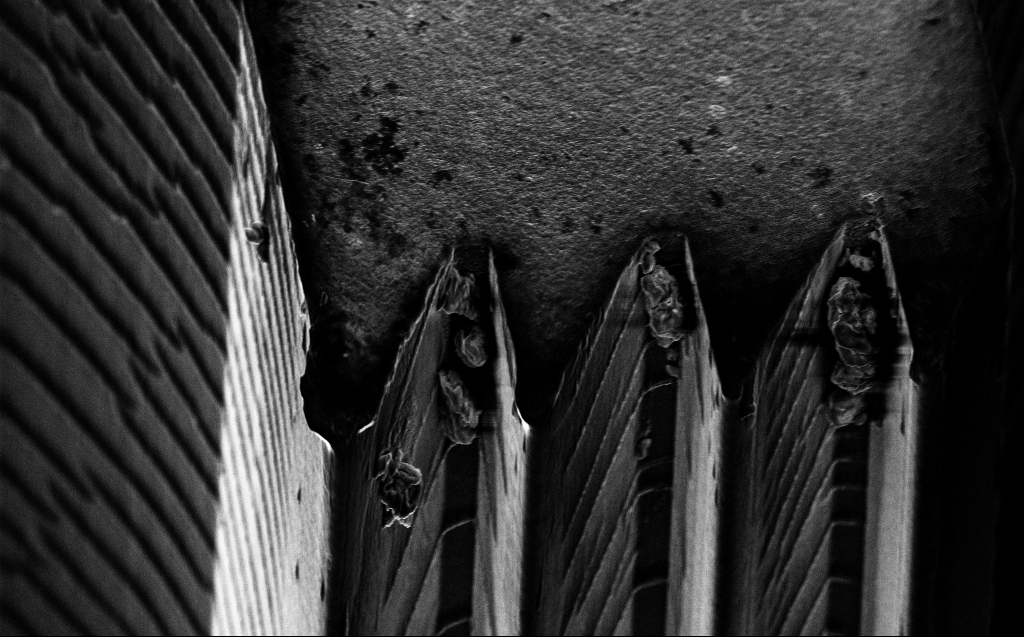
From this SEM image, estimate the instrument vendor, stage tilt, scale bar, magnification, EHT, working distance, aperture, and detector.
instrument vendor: Zeiss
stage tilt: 45°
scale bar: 10000 nm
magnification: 6.55 K X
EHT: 5 kV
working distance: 4 mm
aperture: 30 µm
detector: InLens